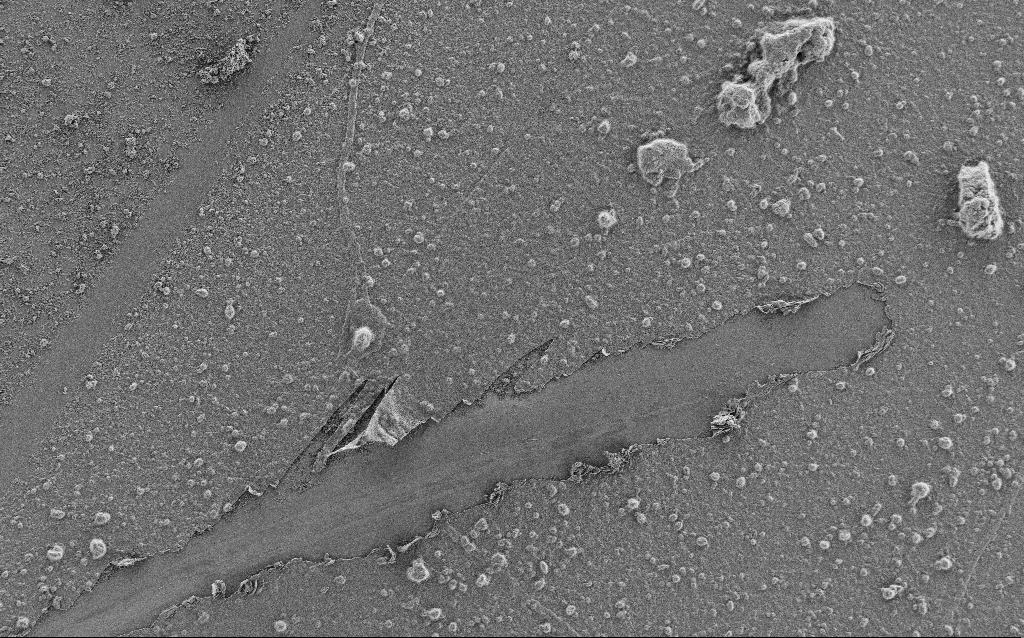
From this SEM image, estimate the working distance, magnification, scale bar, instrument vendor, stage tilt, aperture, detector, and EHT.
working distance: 4 mm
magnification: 1 K X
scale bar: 20000 nm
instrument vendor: Zeiss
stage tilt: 0°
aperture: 30 µm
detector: SE2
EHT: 1 kV